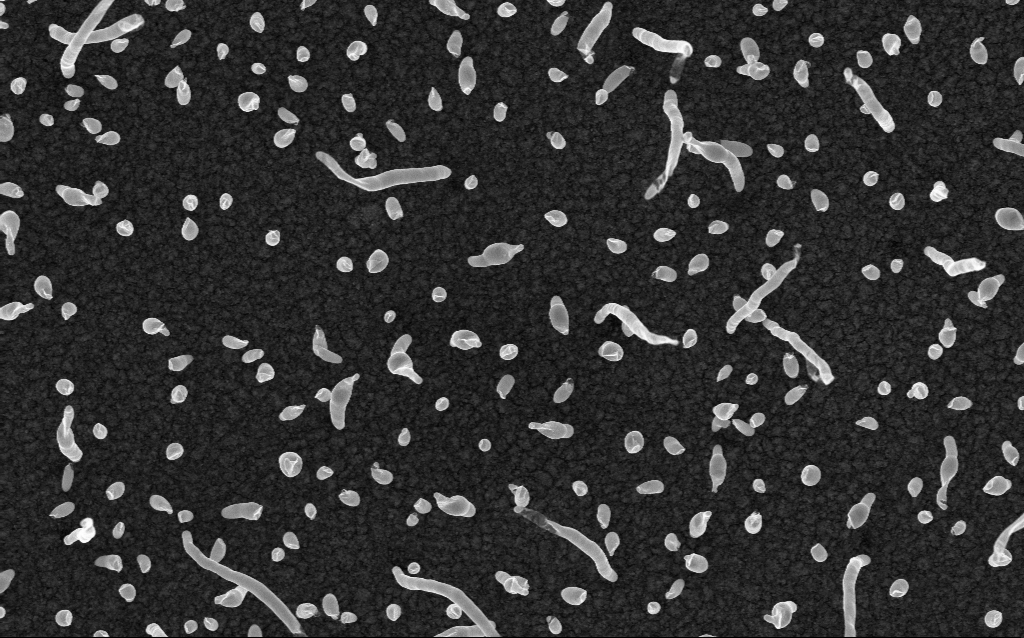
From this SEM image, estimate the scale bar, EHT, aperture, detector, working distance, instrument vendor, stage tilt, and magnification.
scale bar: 1000 nm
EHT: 5 kV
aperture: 30 µm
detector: InLens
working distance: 2.1 mm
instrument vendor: Zeiss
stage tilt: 0°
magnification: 50 K X